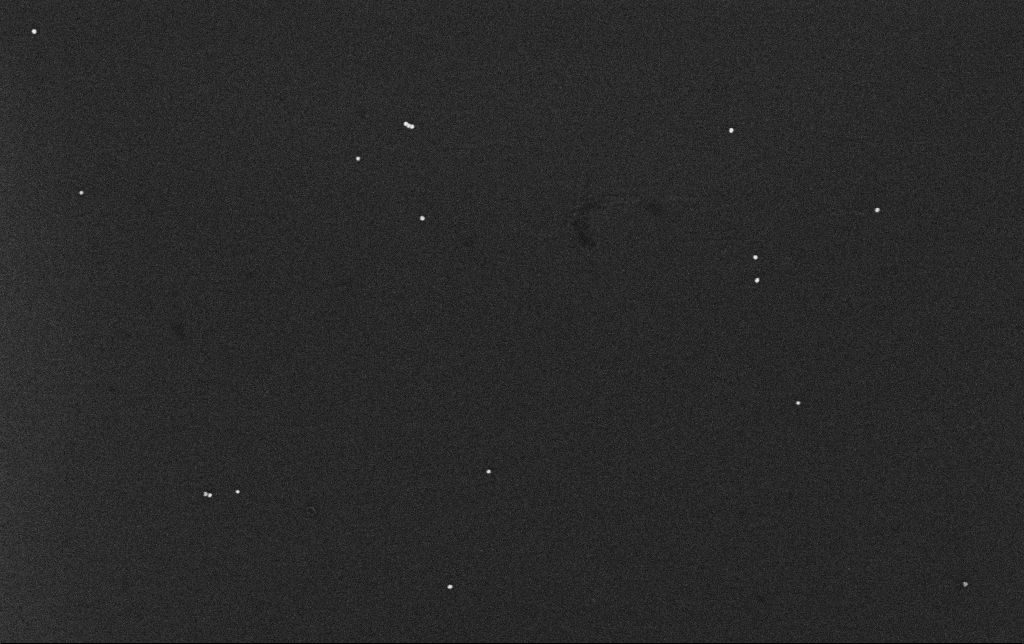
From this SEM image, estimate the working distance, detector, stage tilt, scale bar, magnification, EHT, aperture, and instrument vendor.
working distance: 3.2 mm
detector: InLens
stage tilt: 0°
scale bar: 200 nm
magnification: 100 K X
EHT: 10 kV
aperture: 30 µm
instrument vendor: Zeiss